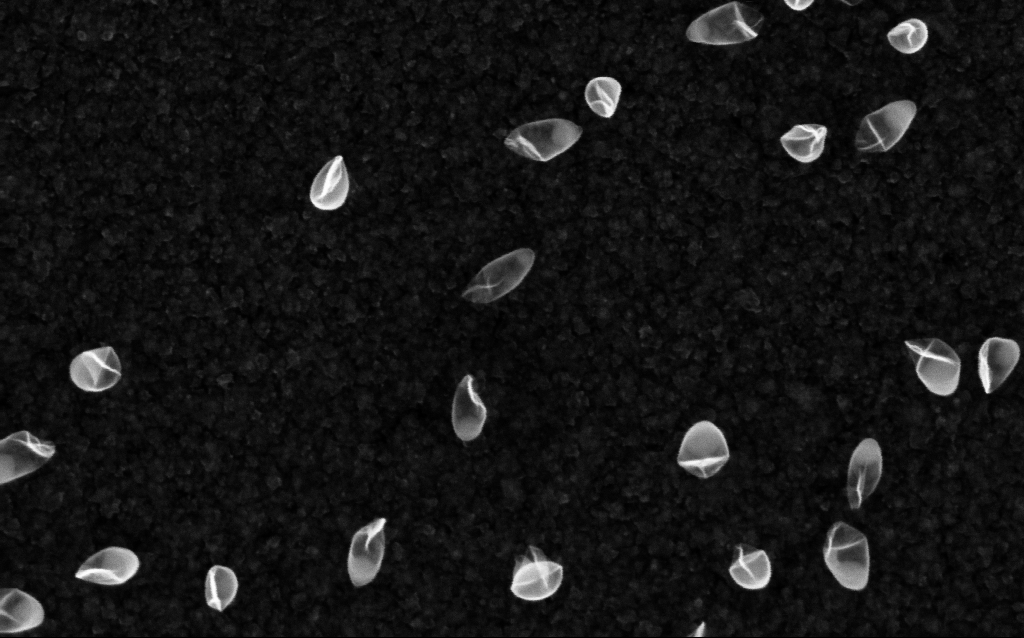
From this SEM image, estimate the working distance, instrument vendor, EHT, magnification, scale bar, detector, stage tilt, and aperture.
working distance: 2.1 mm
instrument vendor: Zeiss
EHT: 5 kV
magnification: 200 K X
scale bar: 100 nm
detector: InLens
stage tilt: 0°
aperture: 30 µm